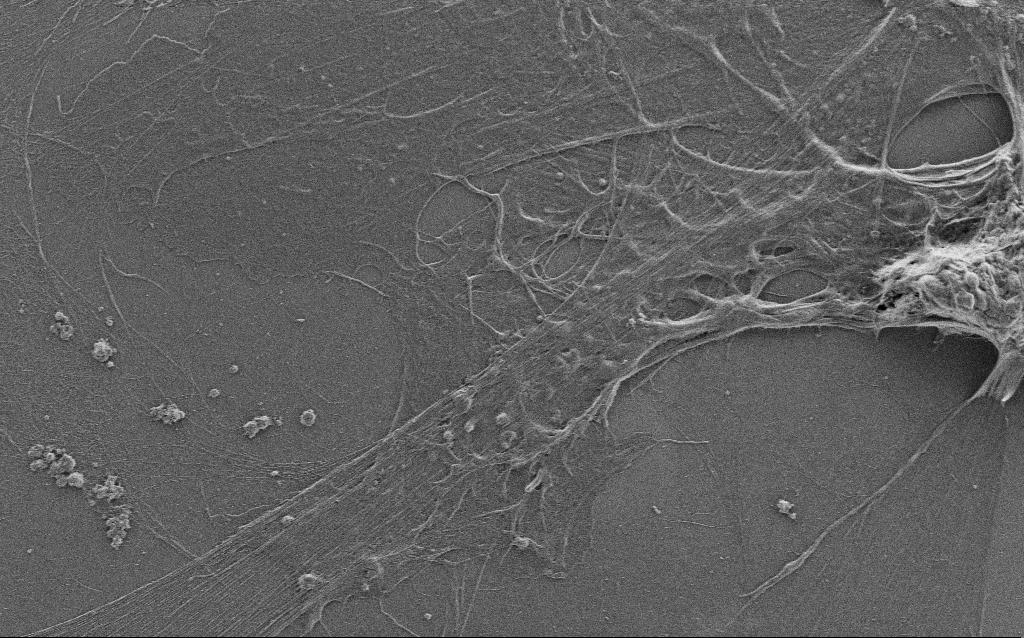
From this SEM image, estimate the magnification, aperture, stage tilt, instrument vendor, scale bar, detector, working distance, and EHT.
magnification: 5 K X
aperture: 30 µm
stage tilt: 0°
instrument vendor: Zeiss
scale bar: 10000 nm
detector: SE2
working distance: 4 mm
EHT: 0.9 kV